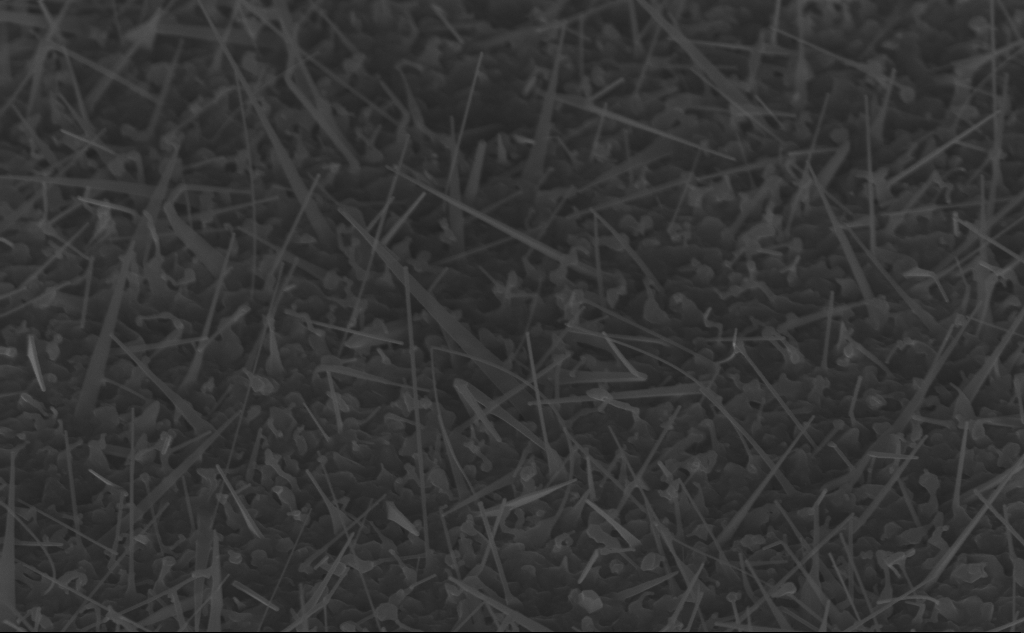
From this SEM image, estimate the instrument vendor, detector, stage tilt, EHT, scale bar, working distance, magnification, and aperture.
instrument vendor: Zeiss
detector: InLens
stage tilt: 45°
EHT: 10 kV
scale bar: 1000 nm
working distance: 8 mm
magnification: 40 K X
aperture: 30 µm